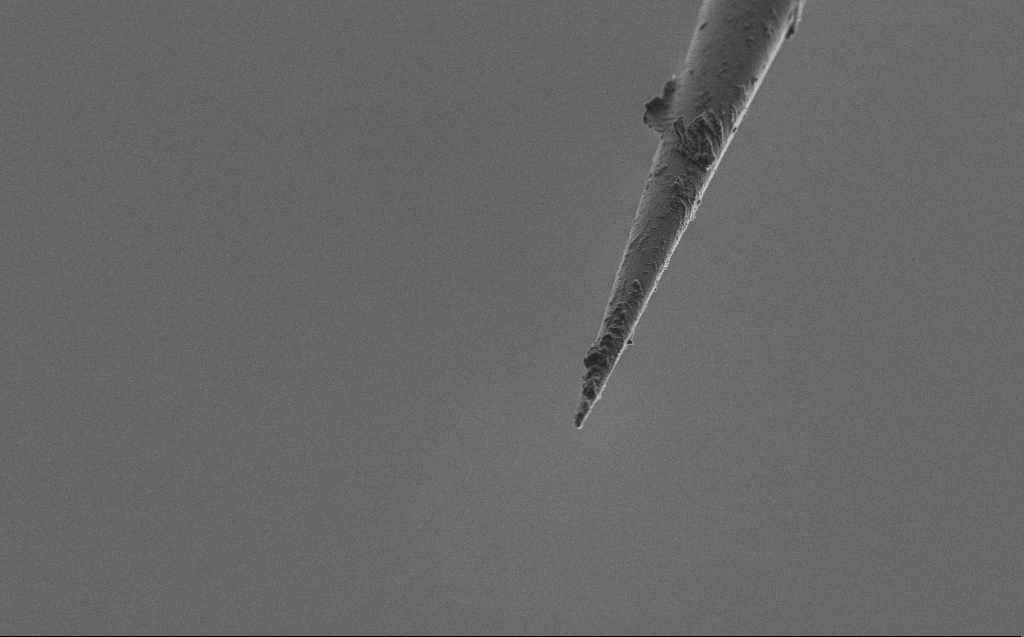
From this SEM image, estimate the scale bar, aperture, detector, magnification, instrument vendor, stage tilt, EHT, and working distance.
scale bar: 10000 nm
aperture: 30 µm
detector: SE2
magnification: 5 K X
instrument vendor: Zeiss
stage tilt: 45°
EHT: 2 kV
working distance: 3 mm